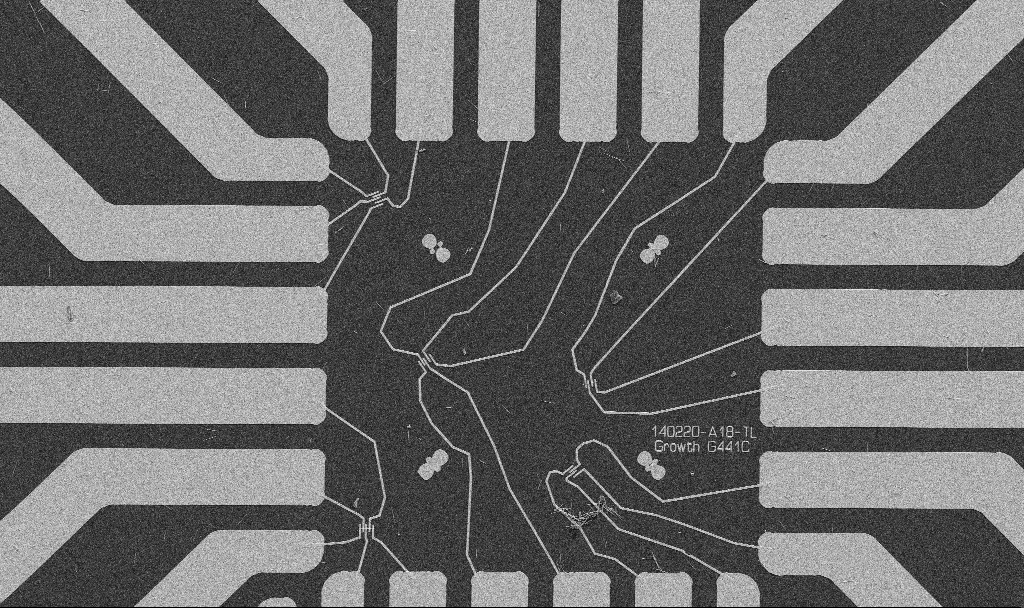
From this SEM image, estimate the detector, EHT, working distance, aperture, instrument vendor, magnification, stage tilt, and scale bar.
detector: SE2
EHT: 5 kV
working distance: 10.7 mm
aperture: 30 µm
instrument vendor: Zeiss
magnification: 1 K X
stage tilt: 0°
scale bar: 20000 nm